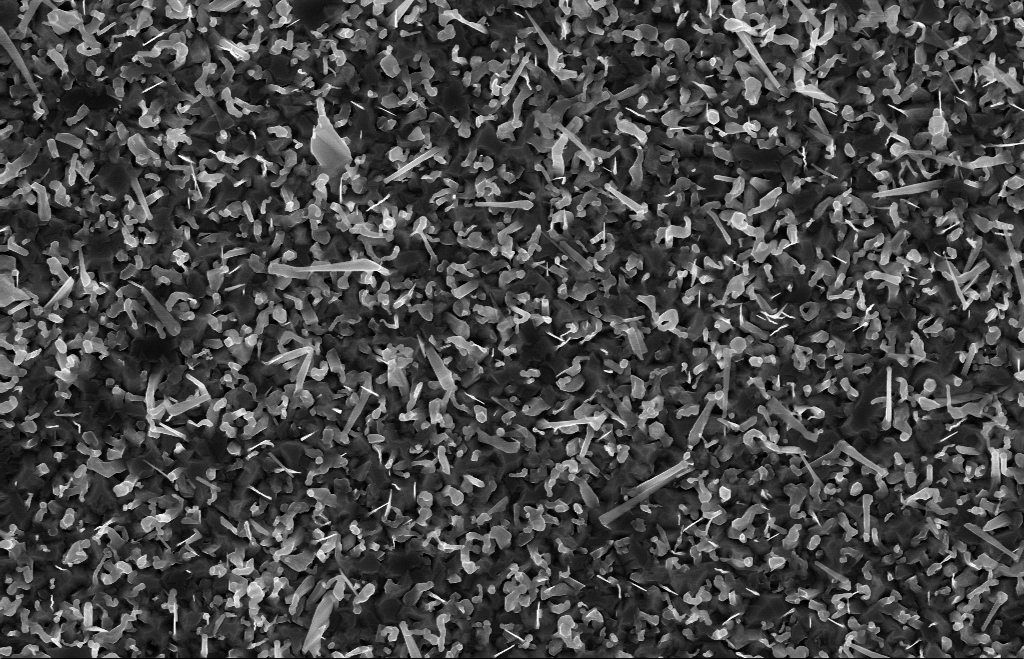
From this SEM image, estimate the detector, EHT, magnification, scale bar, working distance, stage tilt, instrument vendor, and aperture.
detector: InLens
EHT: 10 kV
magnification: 20 K X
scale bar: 1000 nm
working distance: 9 mm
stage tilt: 0°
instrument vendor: Zeiss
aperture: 30 µm